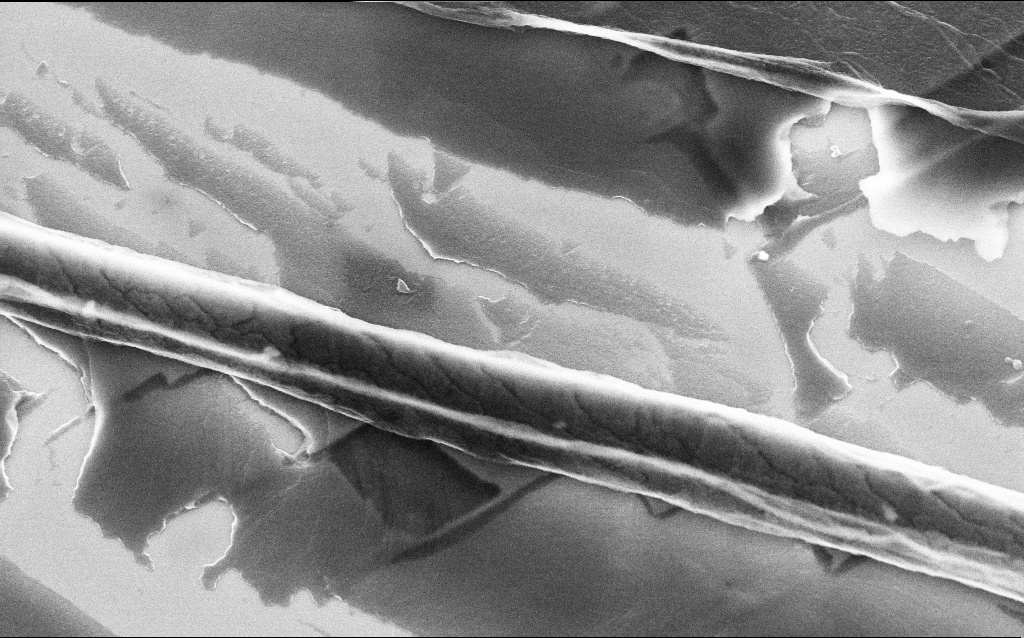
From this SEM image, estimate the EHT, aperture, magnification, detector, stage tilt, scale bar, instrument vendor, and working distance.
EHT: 5 kV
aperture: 30 µm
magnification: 28.72 K X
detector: InLens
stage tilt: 20°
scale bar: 1000 nm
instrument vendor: Zeiss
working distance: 3.7 mm